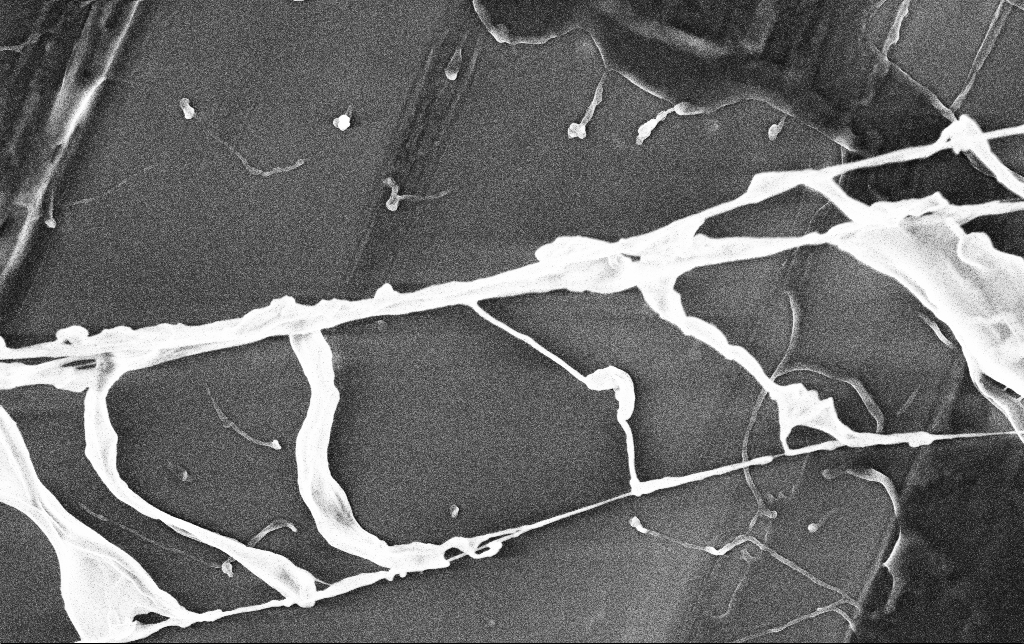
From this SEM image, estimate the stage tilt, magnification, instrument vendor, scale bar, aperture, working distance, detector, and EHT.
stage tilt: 0°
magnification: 23.28 K X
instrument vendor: Zeiss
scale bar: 1000 nm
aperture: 30 µm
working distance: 3.5 mm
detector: InLens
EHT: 3 kV